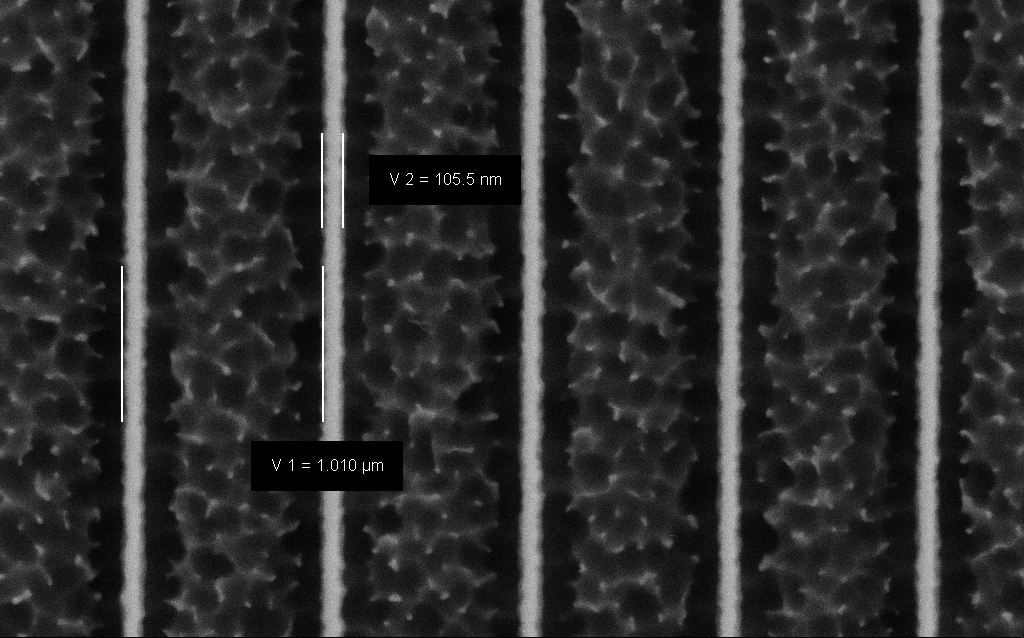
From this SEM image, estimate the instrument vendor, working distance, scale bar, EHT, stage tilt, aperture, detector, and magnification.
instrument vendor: Zeiss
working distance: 5 mm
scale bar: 200 nm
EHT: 3 kV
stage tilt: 0°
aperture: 30 µm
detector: SE2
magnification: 73.1 K X